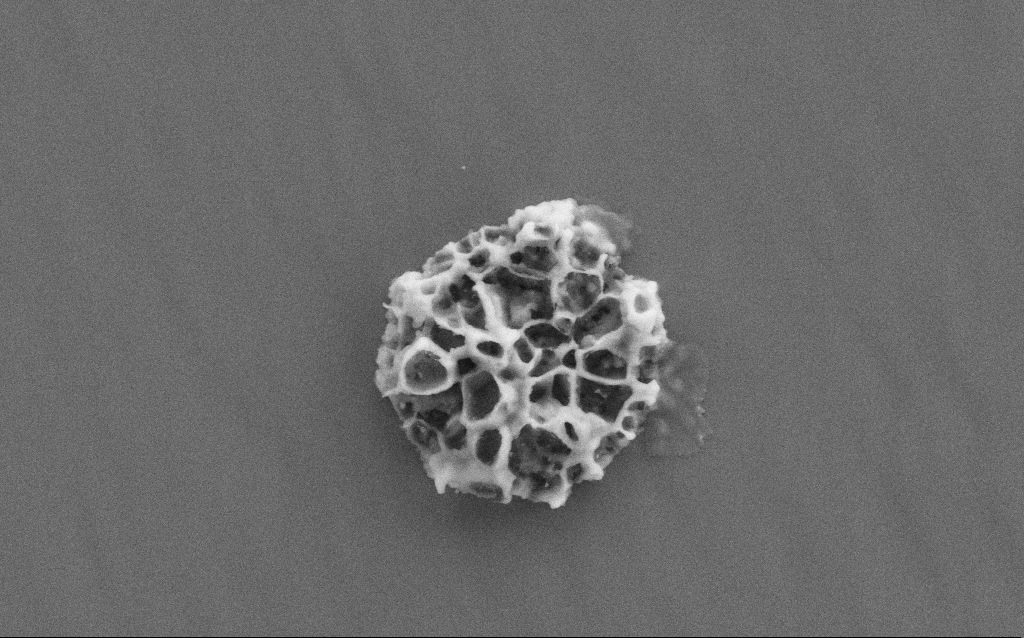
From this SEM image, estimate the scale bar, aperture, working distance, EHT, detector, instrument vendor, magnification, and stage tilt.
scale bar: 1000 nm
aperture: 30 µm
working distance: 2 mm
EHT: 10 kV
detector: SE2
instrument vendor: Zeiss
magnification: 41.24 K X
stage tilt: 0°